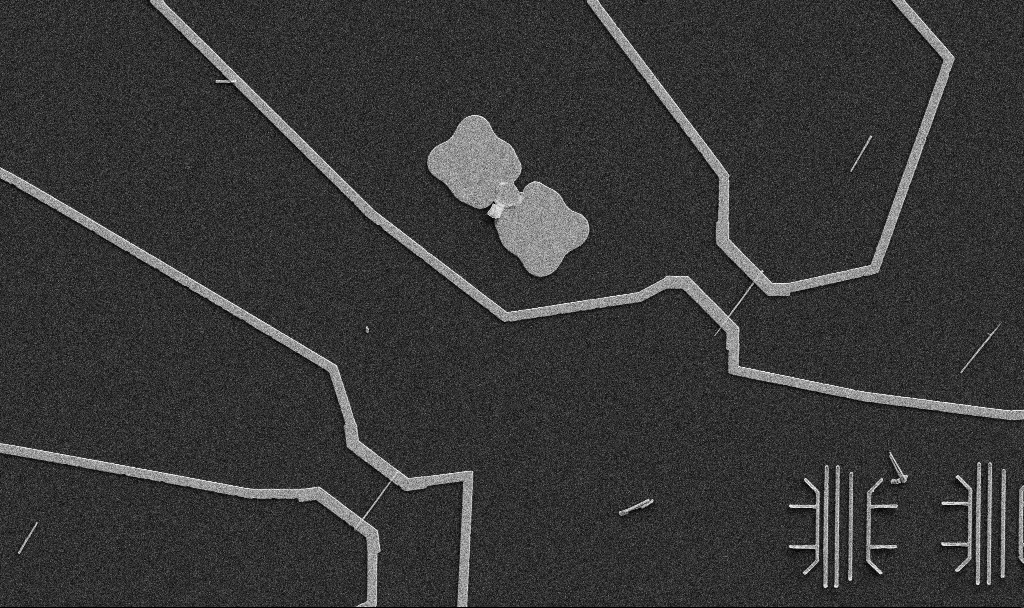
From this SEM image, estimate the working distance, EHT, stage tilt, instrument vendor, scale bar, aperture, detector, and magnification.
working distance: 10.7 mm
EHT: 5 kV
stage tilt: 0°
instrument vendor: Zeiss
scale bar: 10000 nm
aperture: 30 µm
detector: SE2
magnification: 5 K X